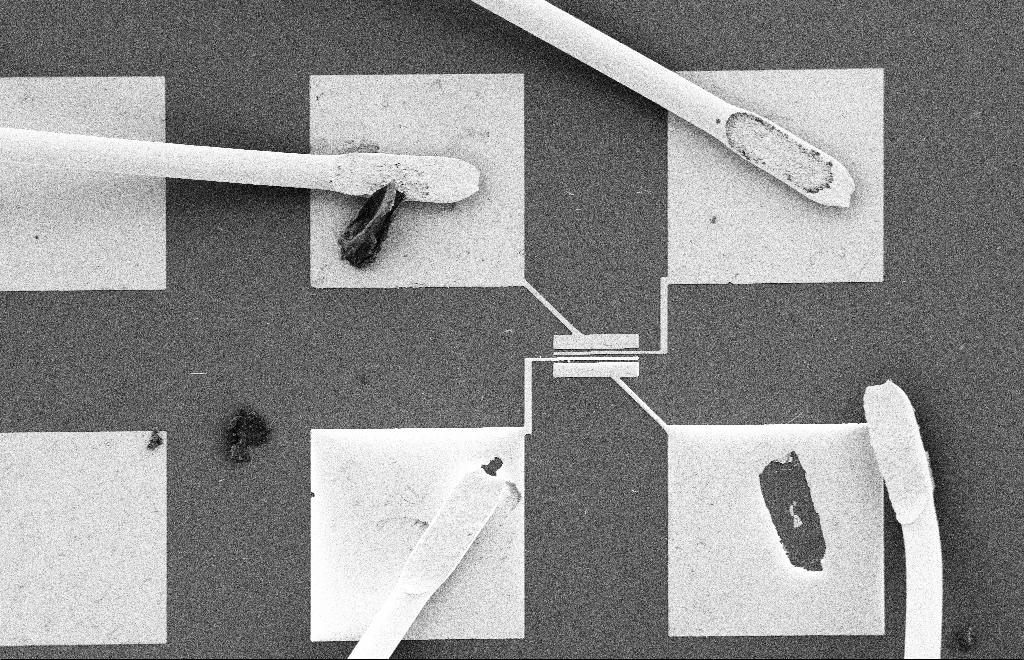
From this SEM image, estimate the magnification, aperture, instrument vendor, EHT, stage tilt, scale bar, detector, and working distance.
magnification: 0.517 K X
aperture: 20 µm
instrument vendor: Zeiss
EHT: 2 kV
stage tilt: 0°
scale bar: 20000 nm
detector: SE2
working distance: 10 mm